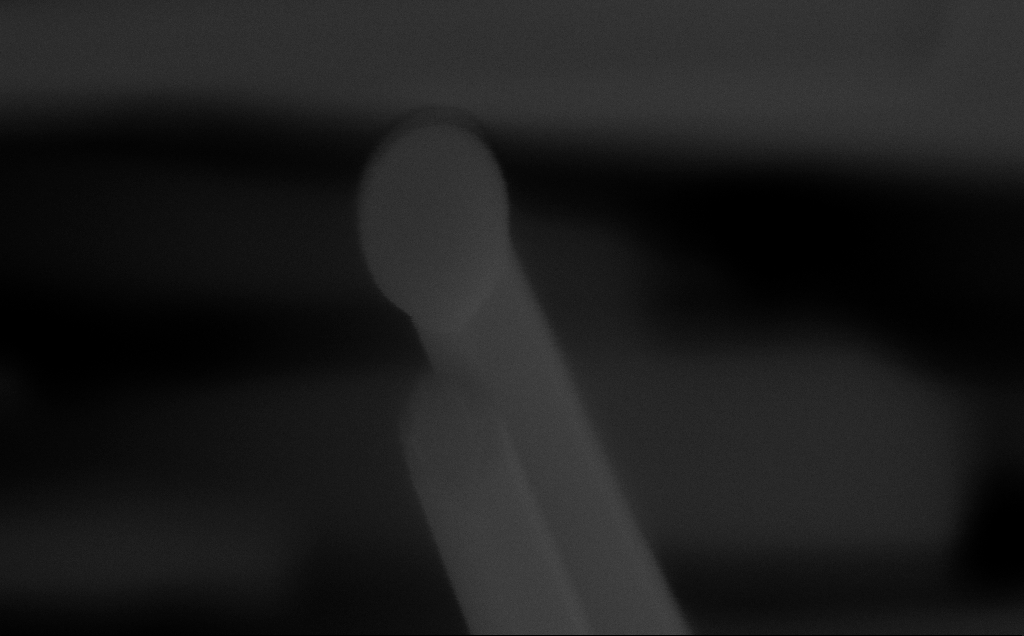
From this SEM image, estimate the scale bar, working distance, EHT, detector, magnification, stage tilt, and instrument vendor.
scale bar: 100 nm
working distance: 6 mm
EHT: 10 kV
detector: InLens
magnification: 516.81 K X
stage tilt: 0°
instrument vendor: Zeiss